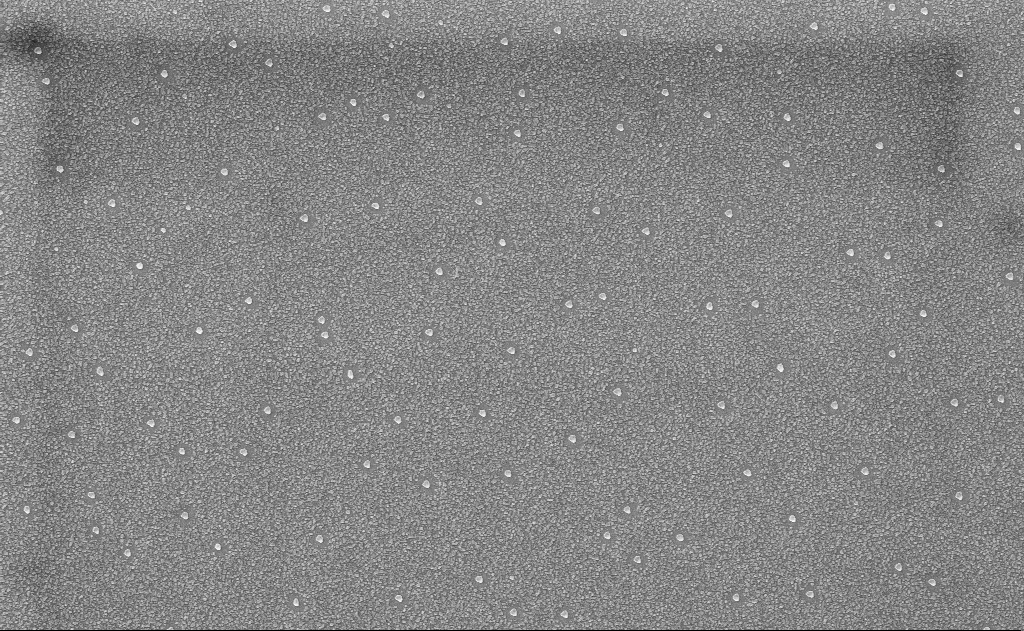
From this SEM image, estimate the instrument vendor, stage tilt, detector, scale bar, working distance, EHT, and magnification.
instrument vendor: Zeiss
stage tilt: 0°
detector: InLens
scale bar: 2000 nm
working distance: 15 mm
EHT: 10 kV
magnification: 20 K X